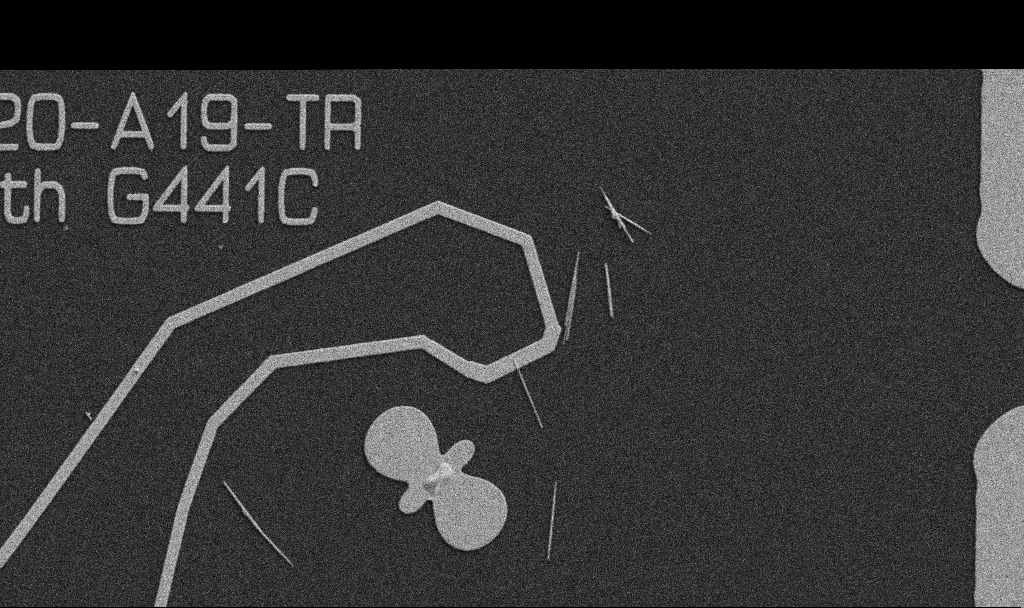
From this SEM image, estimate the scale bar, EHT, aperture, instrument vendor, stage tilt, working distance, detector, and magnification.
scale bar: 10000 nm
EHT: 5 kV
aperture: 30 µm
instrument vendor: Zeiss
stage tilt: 0°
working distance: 10.7 mm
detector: SE2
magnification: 5 K X